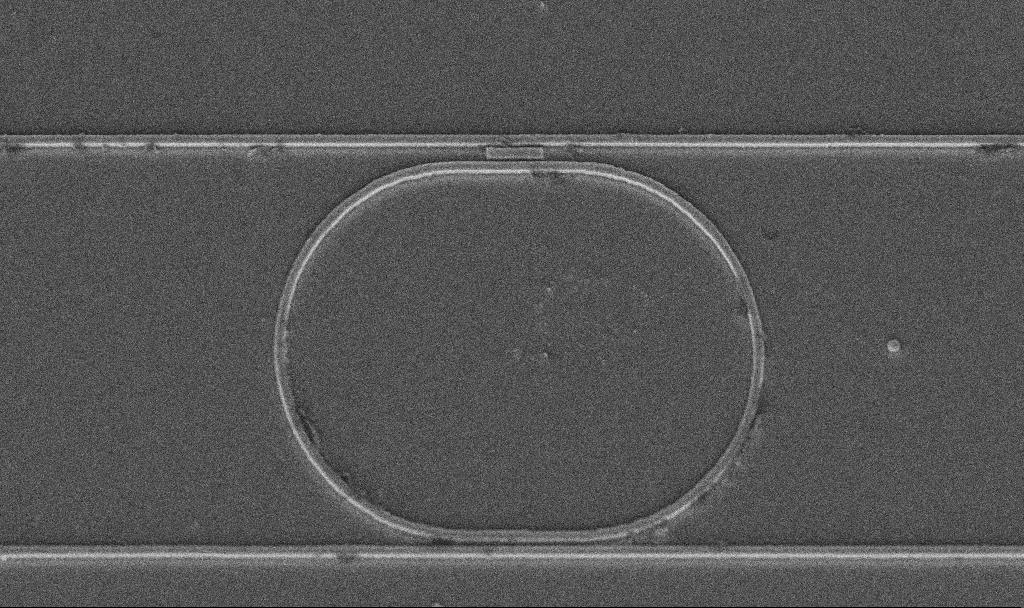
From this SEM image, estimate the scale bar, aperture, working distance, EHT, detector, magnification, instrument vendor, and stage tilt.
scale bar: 10000 nm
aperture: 30 µm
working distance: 9.4 mm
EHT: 5 kV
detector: InLens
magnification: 6.91 K X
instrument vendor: Zeiss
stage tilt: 45°